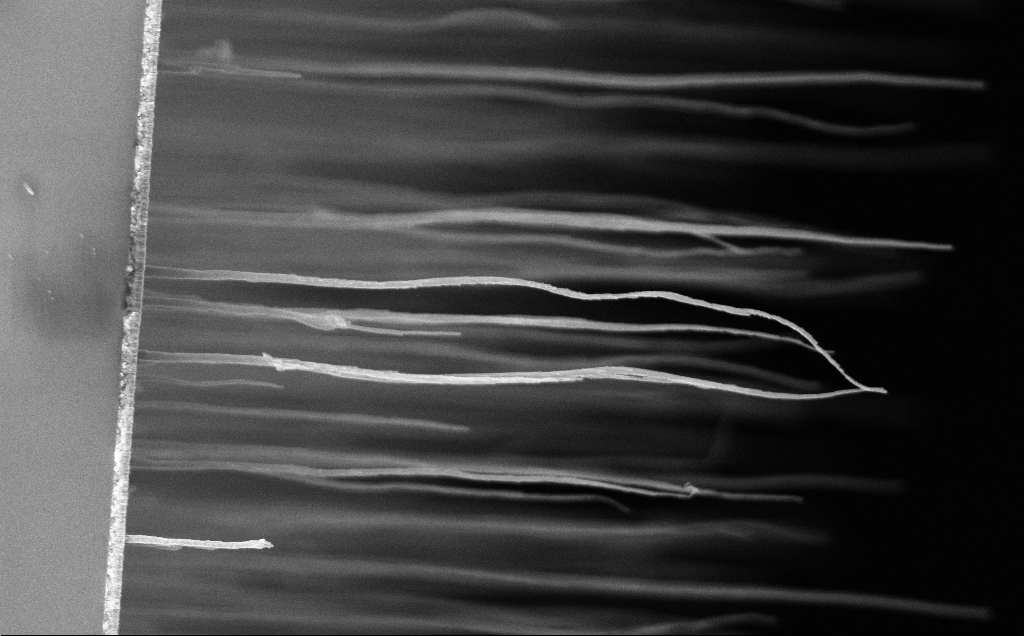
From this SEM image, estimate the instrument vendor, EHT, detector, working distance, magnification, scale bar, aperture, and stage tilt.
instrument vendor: Zeiss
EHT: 5 kV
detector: InLens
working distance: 12 mm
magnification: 23.59 K X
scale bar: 2000 nm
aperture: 30 µm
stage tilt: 0°